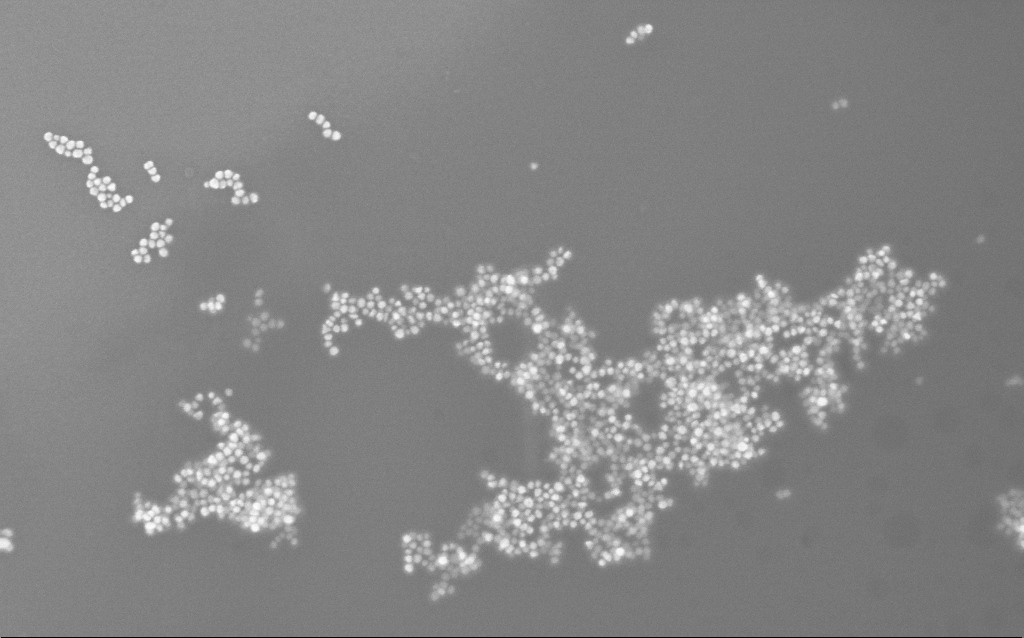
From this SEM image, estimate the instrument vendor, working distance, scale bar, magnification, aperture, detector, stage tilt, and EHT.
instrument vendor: Zeiss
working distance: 3.2 mm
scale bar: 200 nm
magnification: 195.23 K X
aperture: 30 µm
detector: InLens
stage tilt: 0°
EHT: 10 kV